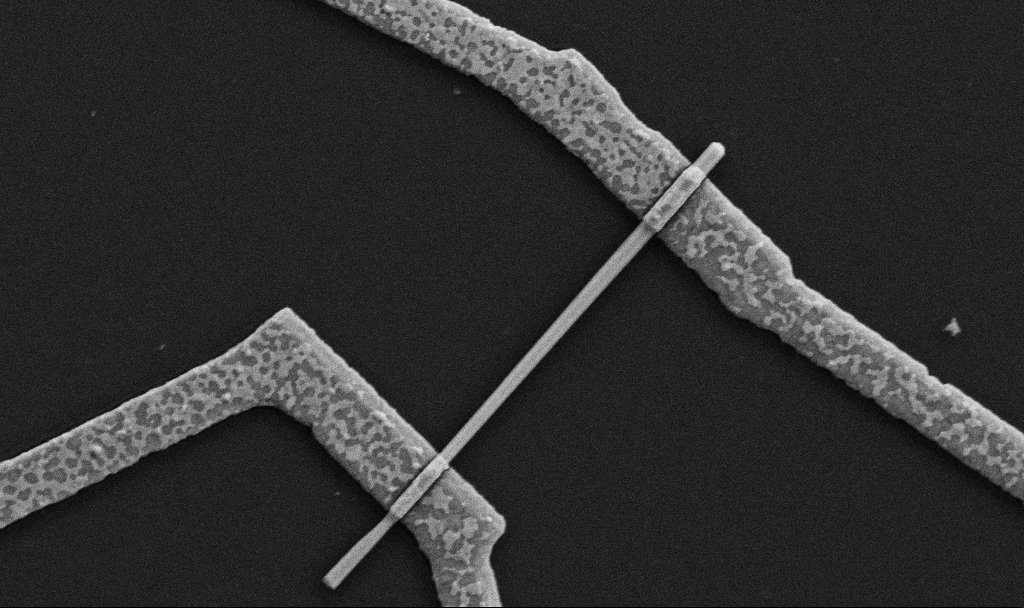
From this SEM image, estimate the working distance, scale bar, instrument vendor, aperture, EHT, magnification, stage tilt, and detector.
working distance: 8.7 mm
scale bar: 1000 nm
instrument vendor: Zeiss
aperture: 30 µm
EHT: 5 kV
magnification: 30 K X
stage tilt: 0°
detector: SE2